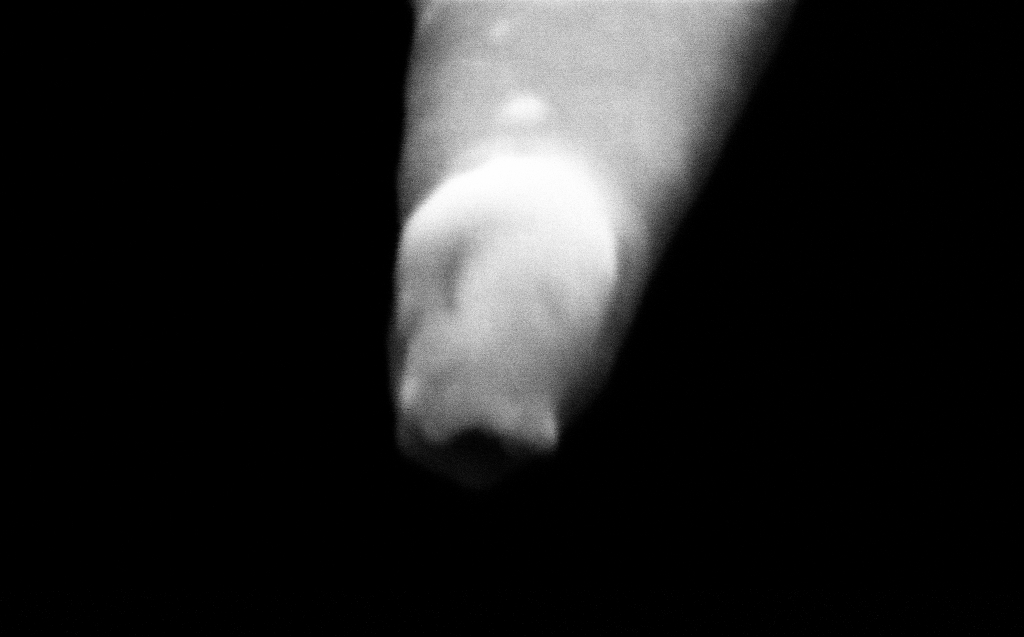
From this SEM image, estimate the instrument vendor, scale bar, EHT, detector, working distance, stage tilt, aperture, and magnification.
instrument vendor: Zeiss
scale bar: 100 nm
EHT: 2 kV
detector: InLens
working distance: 5 mm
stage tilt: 45°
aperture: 30 µm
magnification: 500 K X